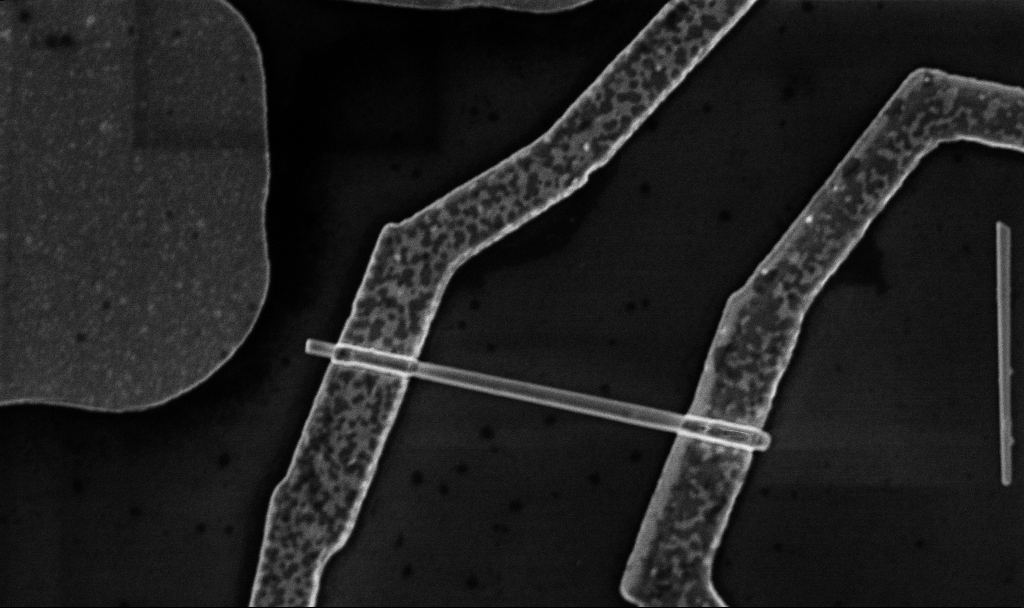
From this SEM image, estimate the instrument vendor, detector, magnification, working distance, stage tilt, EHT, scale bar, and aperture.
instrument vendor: Zeiss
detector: InLens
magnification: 30 K X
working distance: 8.7 mm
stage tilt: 0°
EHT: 5 kV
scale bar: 1000 nm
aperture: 30 µm